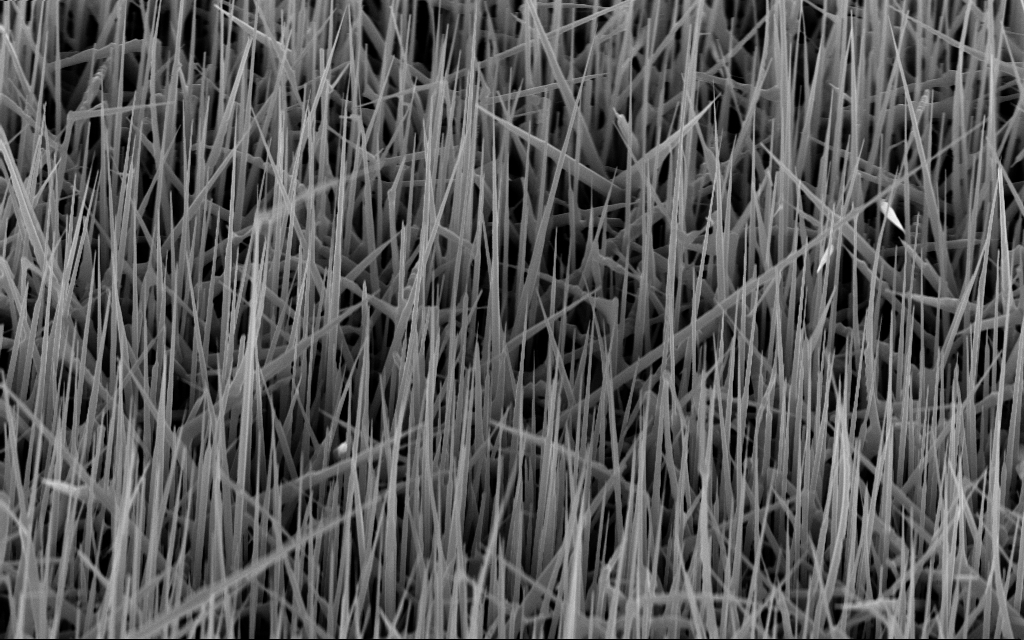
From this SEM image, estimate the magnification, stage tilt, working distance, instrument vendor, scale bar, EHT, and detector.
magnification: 20 K X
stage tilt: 45°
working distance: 7 mm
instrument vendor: Zeiss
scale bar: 1000 nm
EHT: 10 kV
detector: InLens